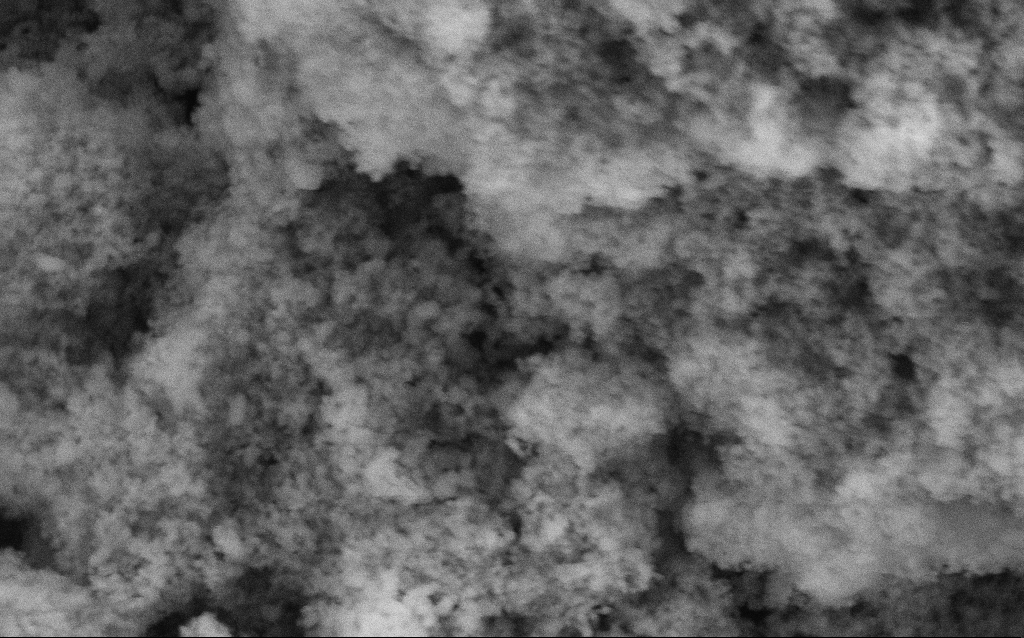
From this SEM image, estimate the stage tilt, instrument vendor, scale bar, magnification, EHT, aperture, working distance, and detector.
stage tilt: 0°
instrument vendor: Zeiss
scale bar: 200 nm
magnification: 114.25 K X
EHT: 5 kV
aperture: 30 µm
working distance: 4.6 mm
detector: SE2